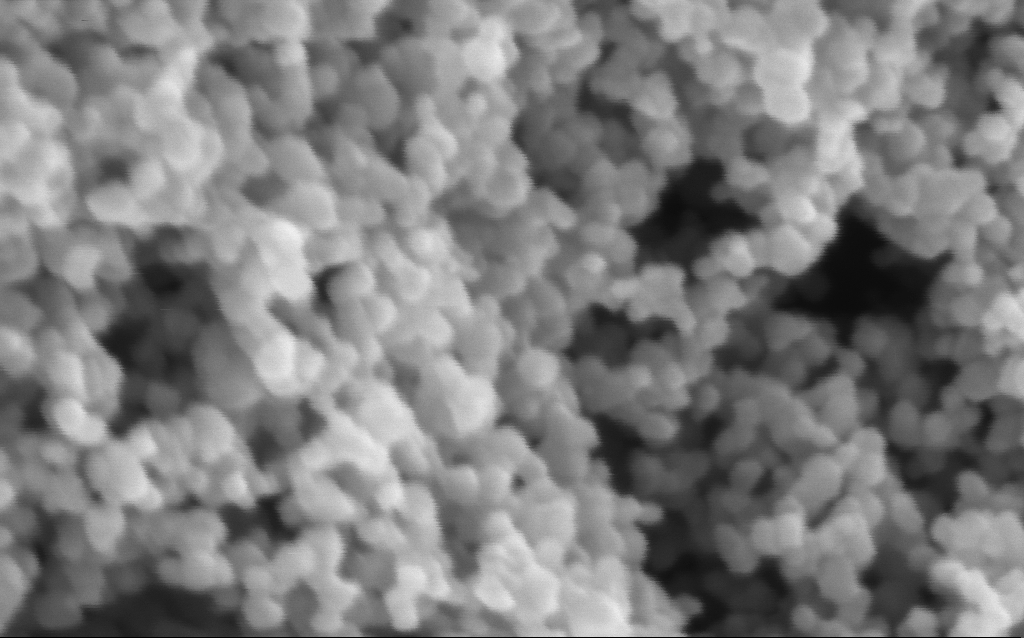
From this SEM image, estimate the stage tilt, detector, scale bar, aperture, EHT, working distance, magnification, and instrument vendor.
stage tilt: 0°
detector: InLens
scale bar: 100 nm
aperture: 30 µm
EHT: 3 kV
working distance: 7.5 mm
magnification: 416 K X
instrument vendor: Zeiss